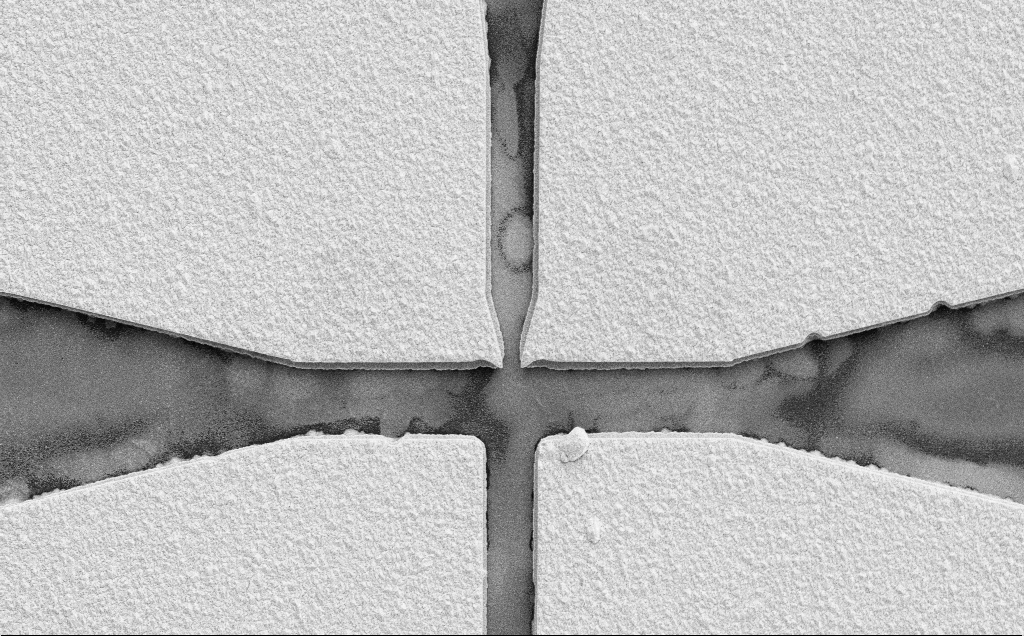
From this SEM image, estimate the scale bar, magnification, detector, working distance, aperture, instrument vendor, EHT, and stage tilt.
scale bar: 20000 nm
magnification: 2.05 K X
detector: SE2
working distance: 14 mm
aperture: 30 µm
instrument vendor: Zeiss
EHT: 10 kV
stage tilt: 0°